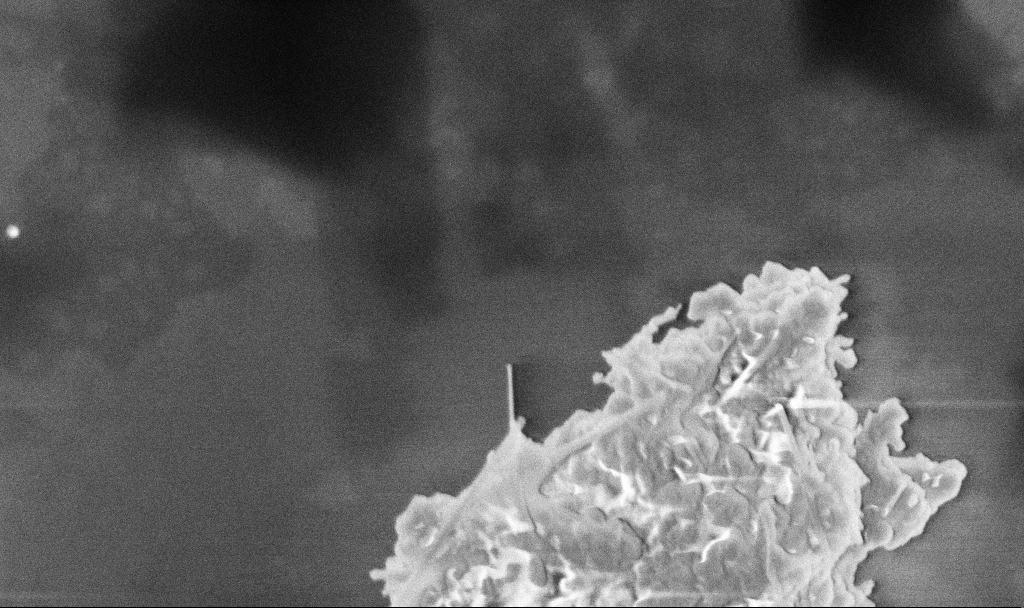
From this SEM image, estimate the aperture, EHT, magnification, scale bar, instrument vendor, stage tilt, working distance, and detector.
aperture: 30 µm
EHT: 3 kV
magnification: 61.27 K X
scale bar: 1000 nm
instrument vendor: Zeiss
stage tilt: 0°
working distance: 3.3 mm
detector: InLens